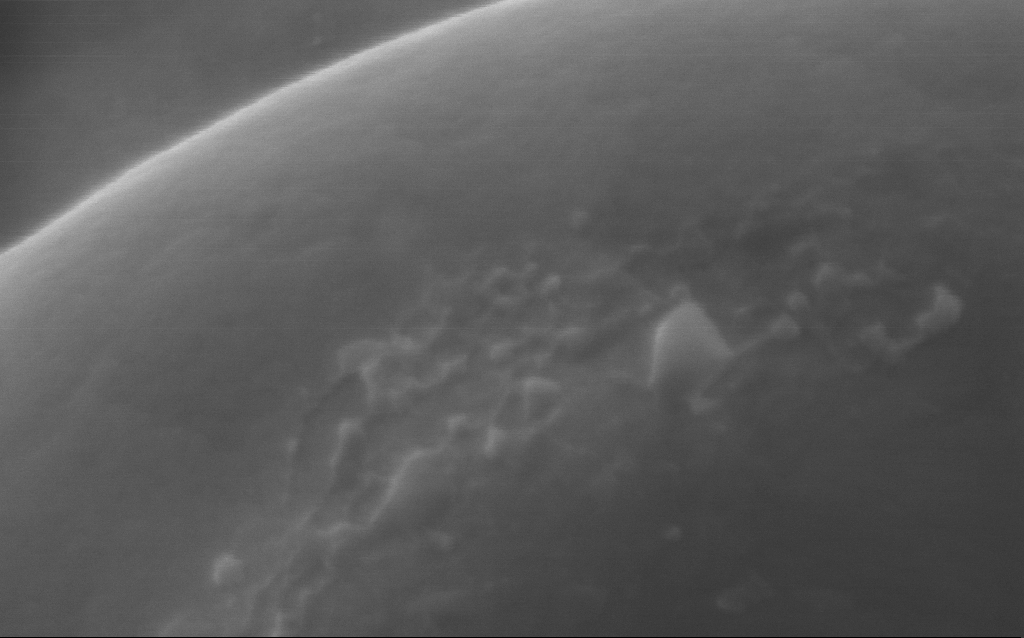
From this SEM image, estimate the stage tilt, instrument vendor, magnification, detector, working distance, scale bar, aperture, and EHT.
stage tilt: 0°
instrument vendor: Zeiss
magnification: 254 K X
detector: InLens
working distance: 3 mm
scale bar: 200 nm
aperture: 30 µm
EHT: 5 kV